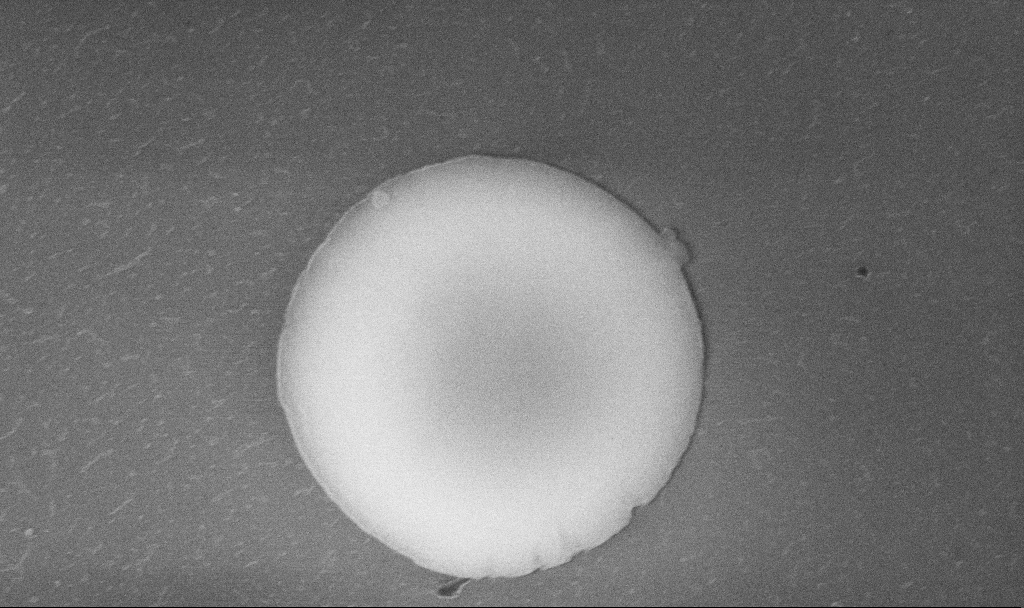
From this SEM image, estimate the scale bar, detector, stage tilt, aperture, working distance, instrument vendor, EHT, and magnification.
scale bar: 1000 nm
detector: InLens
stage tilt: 0°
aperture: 30 µm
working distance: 5.2 mm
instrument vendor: Zeiss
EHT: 10 kV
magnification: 52.01 K X